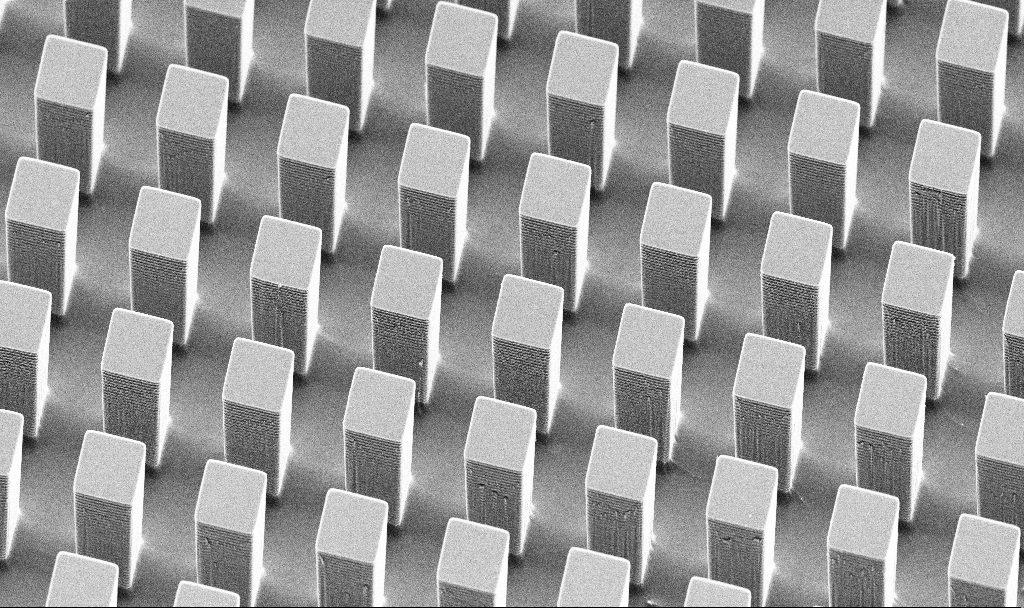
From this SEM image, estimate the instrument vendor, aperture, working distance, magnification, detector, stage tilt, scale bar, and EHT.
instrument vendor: Zeiss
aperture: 30 µm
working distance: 10.1 mm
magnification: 2.86 K X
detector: SE2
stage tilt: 45°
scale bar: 10000 nm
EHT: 5 kV